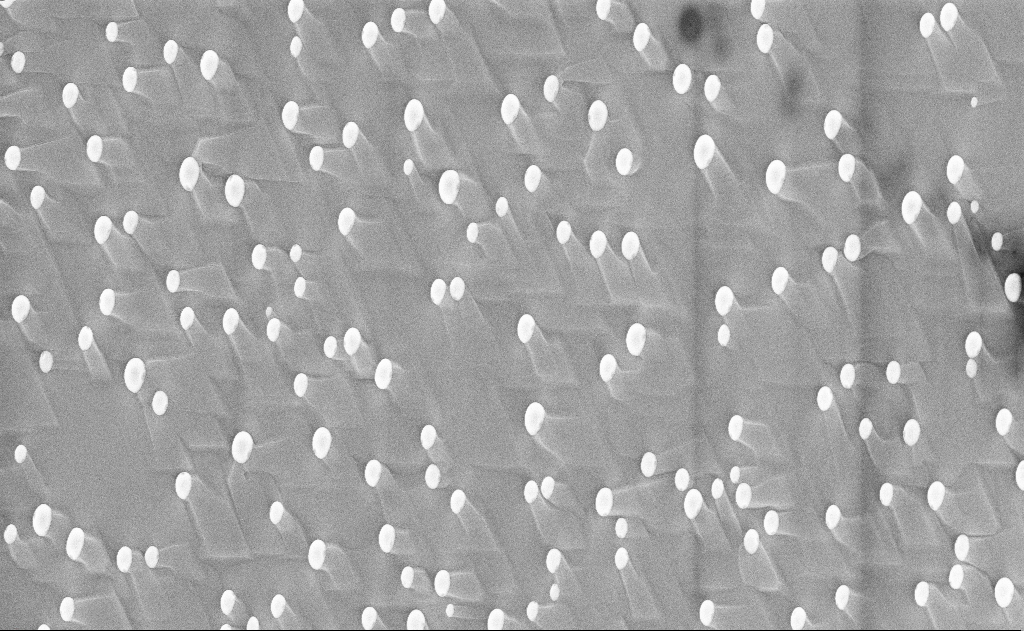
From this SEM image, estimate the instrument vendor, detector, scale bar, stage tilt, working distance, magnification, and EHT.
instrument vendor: Zeiss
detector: InLens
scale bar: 2000 nm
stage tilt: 0°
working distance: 12 mm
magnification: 10 K X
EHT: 10 kV